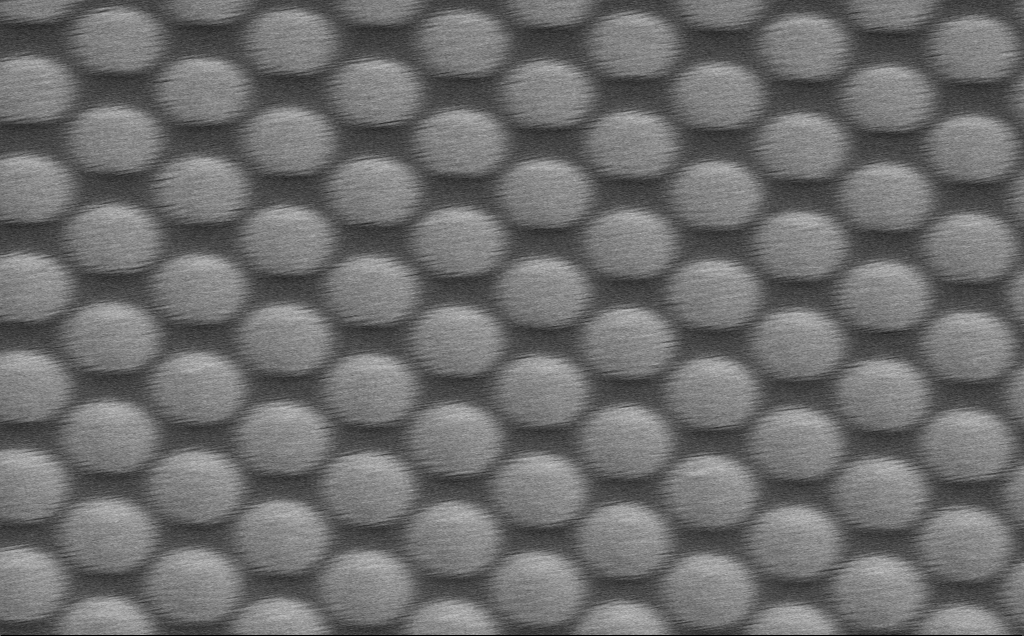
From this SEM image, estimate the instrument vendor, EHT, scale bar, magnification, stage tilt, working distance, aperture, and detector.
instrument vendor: Zeiss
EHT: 5 kV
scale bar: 2000 nm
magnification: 11.99 K X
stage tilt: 0°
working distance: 20 mm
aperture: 30 µm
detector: SE2